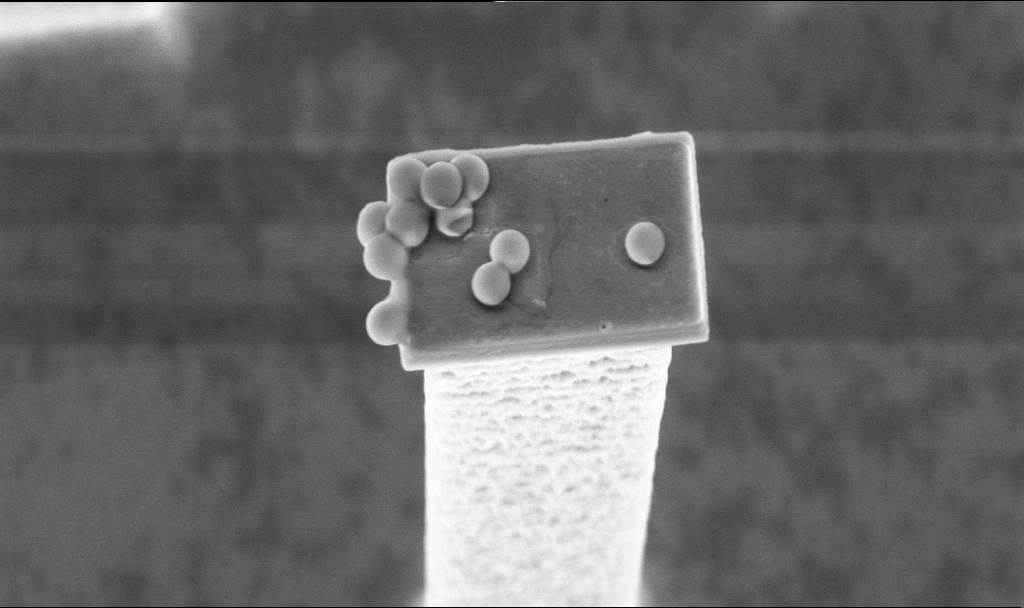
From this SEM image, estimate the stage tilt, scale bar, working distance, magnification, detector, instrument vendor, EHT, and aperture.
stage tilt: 20°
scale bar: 2000 nm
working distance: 3.2 mm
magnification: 24.9 K X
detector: InLens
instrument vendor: Zeiss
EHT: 5 kV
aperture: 30 µm